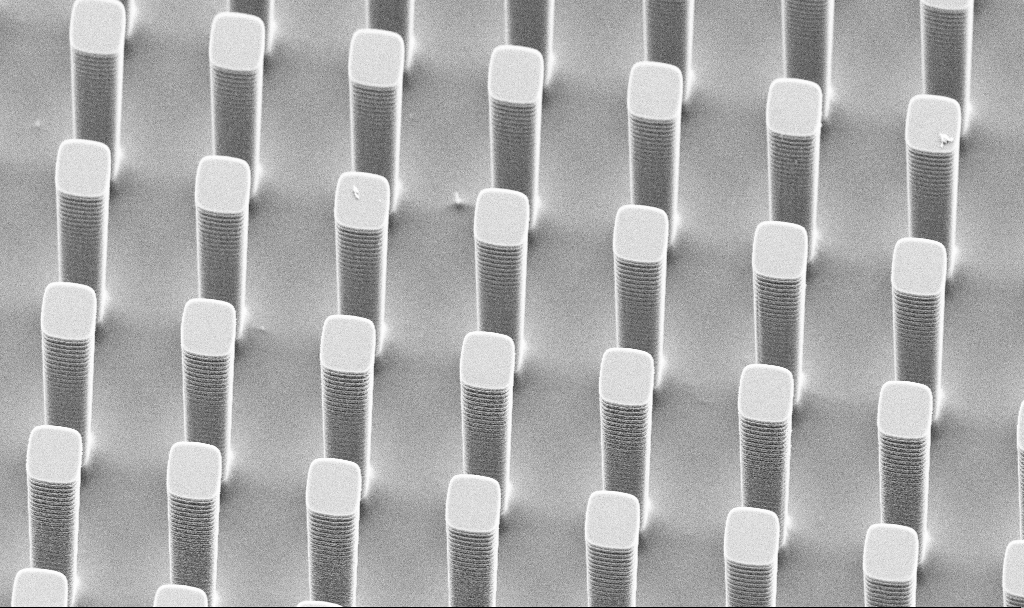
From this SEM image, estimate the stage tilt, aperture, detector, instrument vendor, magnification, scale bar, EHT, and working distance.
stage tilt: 45°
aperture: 30 µm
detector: SE2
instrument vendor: Zeiss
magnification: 4.28 K X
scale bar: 10000 nm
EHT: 5 kV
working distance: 10.1 mm